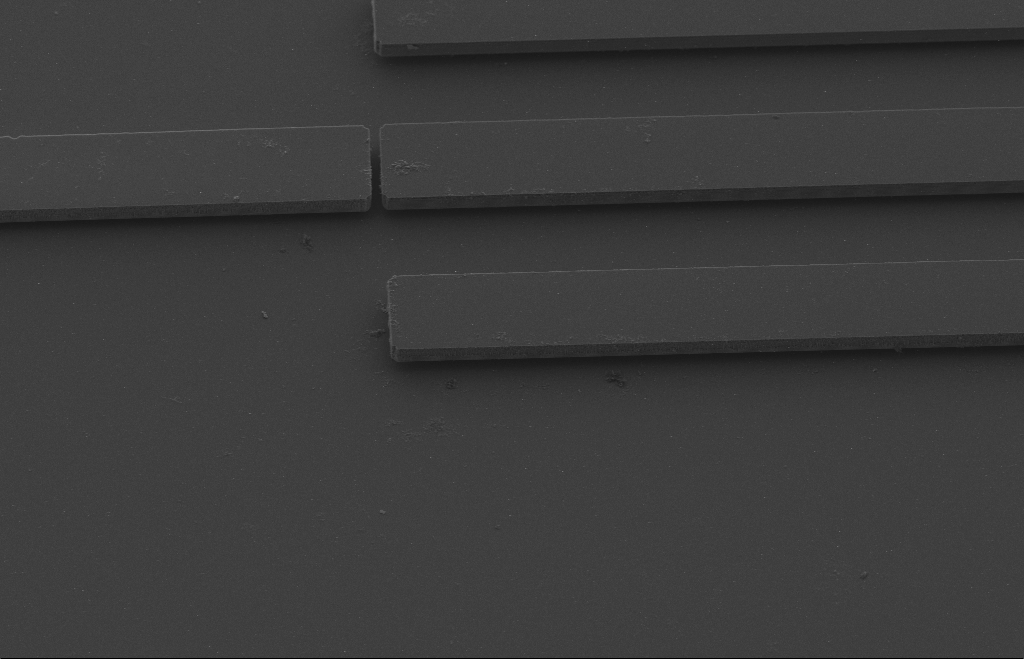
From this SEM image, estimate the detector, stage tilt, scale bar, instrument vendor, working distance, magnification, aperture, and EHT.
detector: SE2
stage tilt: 39°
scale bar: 20000 nm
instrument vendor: Zeiss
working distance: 9 mm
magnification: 1.93 K X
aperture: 20 µm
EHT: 5 kV